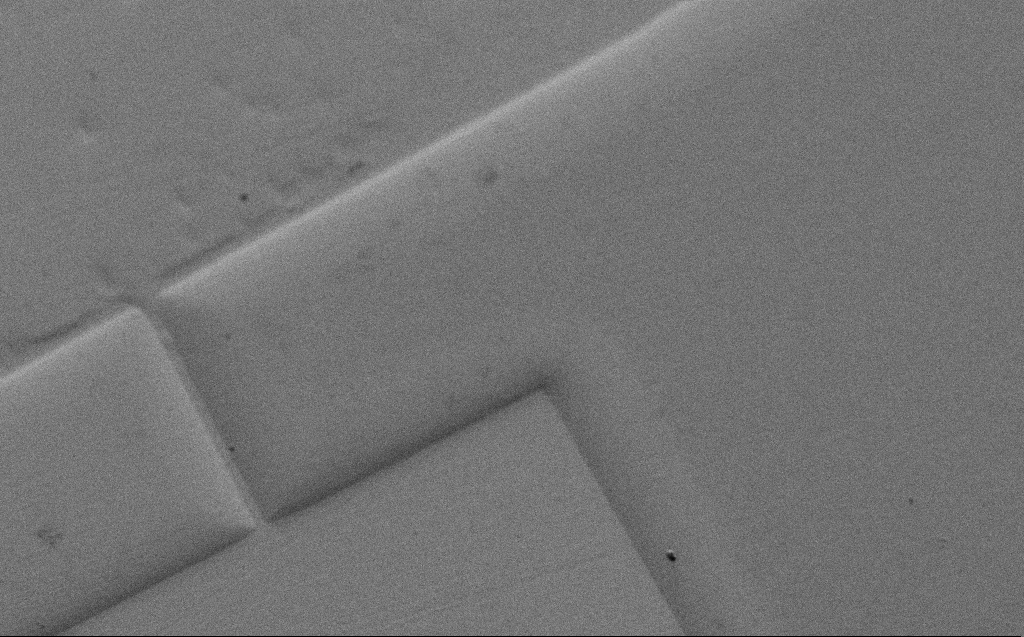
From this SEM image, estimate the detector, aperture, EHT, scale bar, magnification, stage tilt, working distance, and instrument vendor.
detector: SE2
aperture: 30 µm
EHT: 5 kV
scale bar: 20000 nm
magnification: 0.749 K X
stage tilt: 45°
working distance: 7 mm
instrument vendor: Zeiss